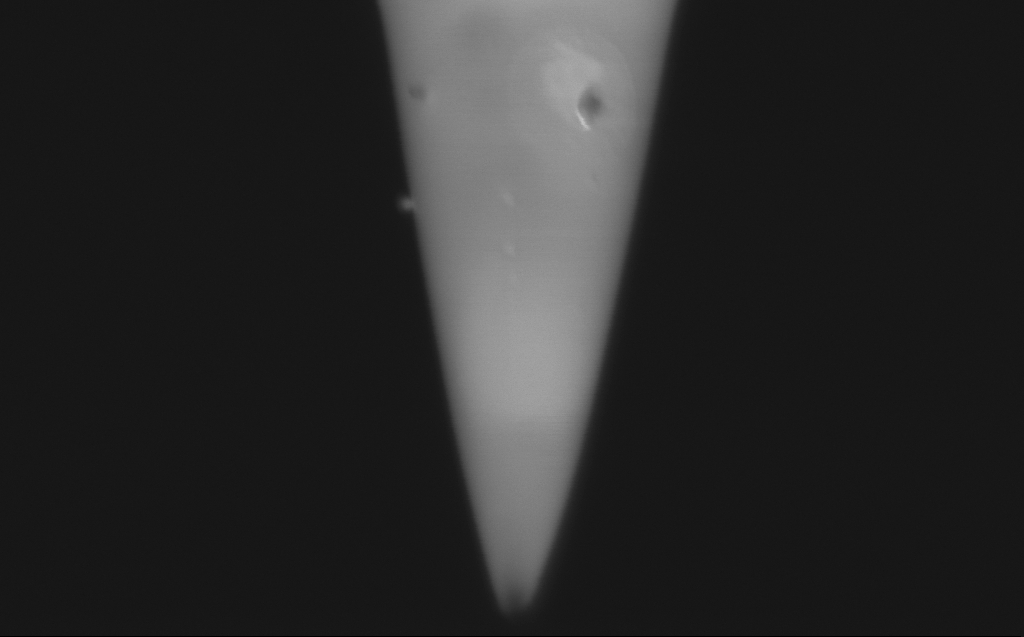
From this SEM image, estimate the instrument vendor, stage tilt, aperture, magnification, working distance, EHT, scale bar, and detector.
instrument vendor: Zeiss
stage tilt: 45.1°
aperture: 30 µm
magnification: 123.74 K X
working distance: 3 mm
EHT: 0.75 kV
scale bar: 200 nm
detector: InLens